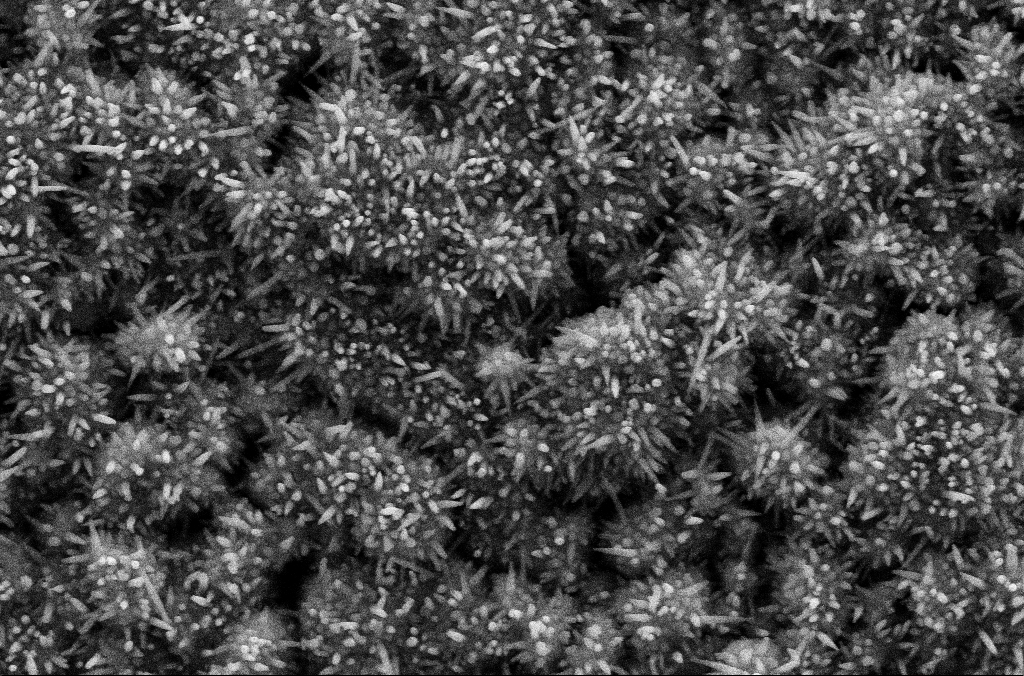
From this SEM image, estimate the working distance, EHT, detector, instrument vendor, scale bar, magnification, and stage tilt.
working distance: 5.2 mm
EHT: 10 kV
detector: SE2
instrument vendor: Zeiss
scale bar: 1000 nm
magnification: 45.76 K X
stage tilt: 0.1°